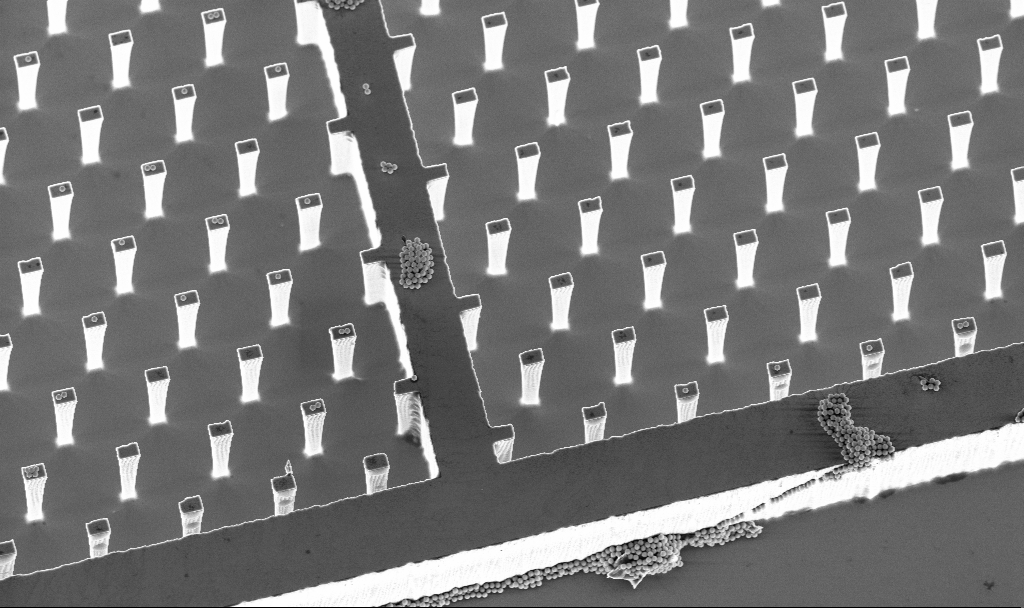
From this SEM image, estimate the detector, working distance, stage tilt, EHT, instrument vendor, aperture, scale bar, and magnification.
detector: InLens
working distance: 4.7 mm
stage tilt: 20°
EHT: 5 kV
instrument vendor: Zeiss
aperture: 30 µm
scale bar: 10000 nm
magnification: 2.93 K X